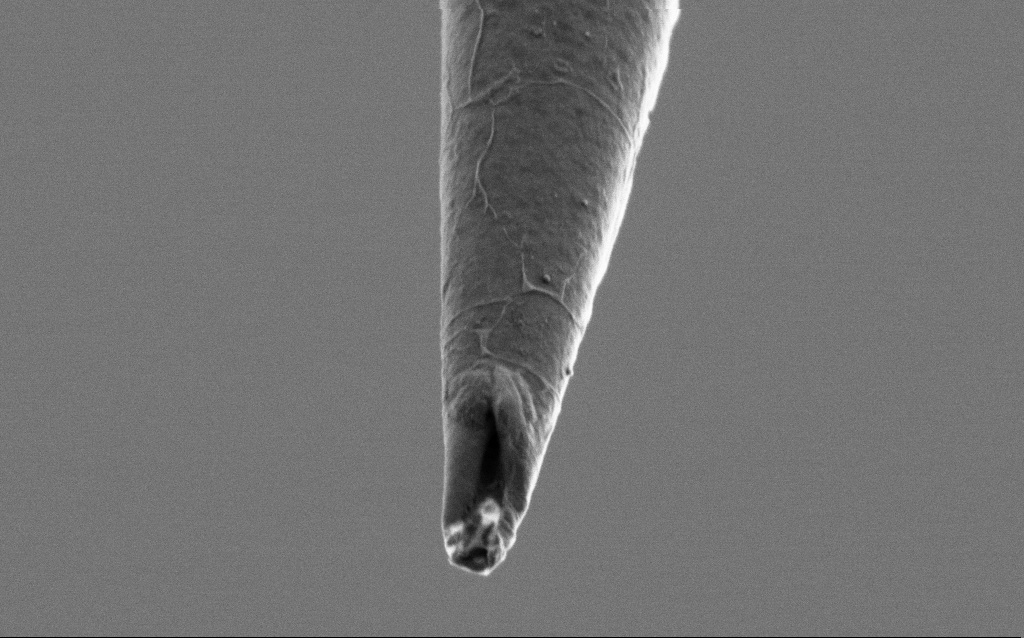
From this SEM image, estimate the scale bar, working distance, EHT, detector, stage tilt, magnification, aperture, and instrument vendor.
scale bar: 1000 nm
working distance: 6 mm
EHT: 1 kV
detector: SE2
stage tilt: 45°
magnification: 42.72 K X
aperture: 30 µm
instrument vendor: Zeiss